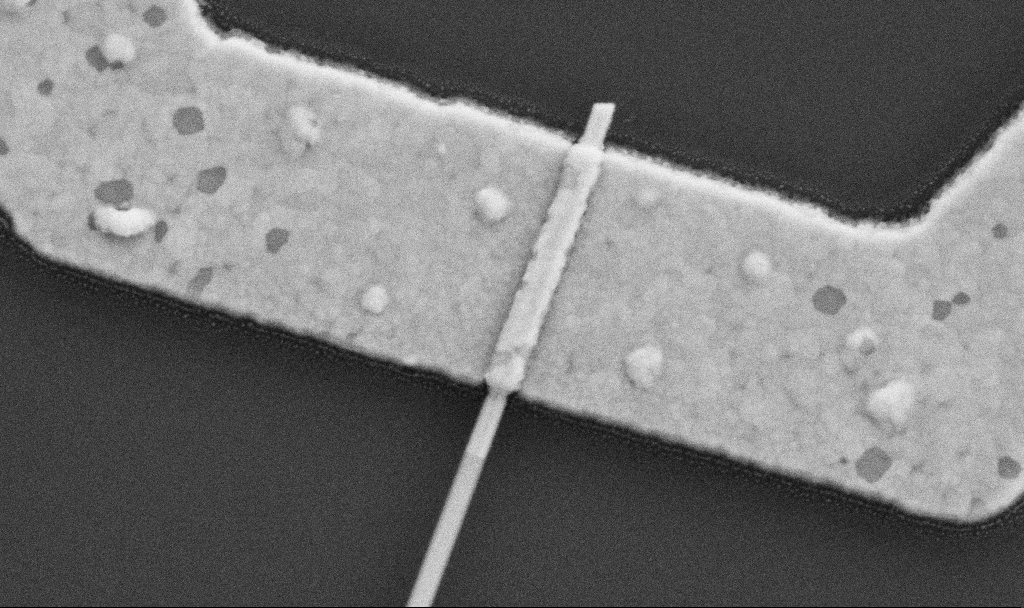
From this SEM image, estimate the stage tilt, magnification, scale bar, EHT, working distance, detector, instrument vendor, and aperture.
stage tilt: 0°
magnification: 100 K X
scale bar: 200 nm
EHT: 5 kV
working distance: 8.7 mm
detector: SE2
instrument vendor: Zeiss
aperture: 30 µm